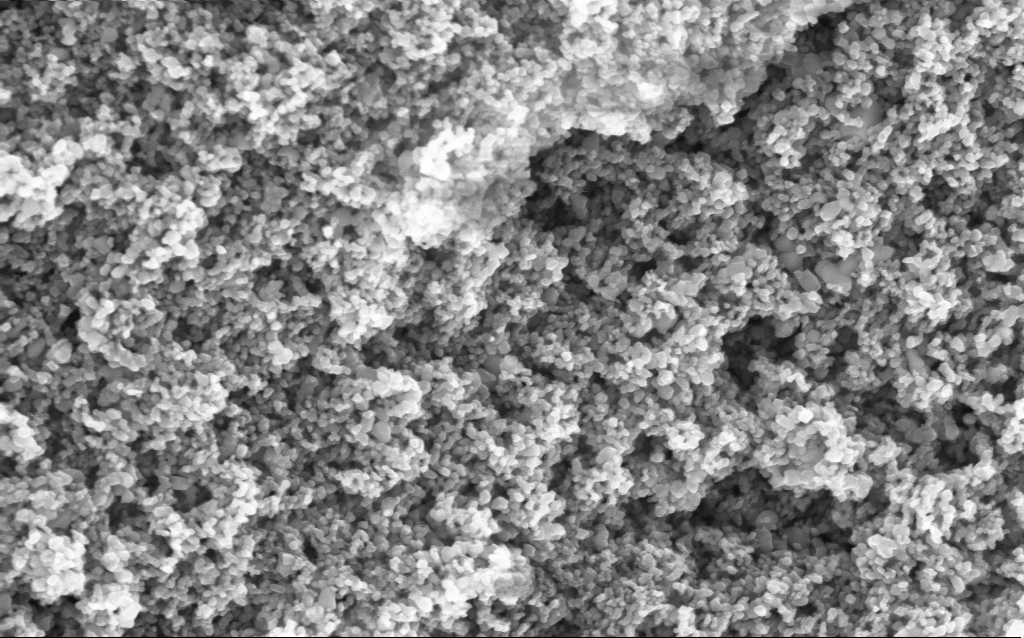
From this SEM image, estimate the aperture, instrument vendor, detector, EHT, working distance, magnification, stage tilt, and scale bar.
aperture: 30 µm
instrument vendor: Zeiss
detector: InLens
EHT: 5 kV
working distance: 4.5 mm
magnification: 114.64 K X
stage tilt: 0°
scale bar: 200 nm